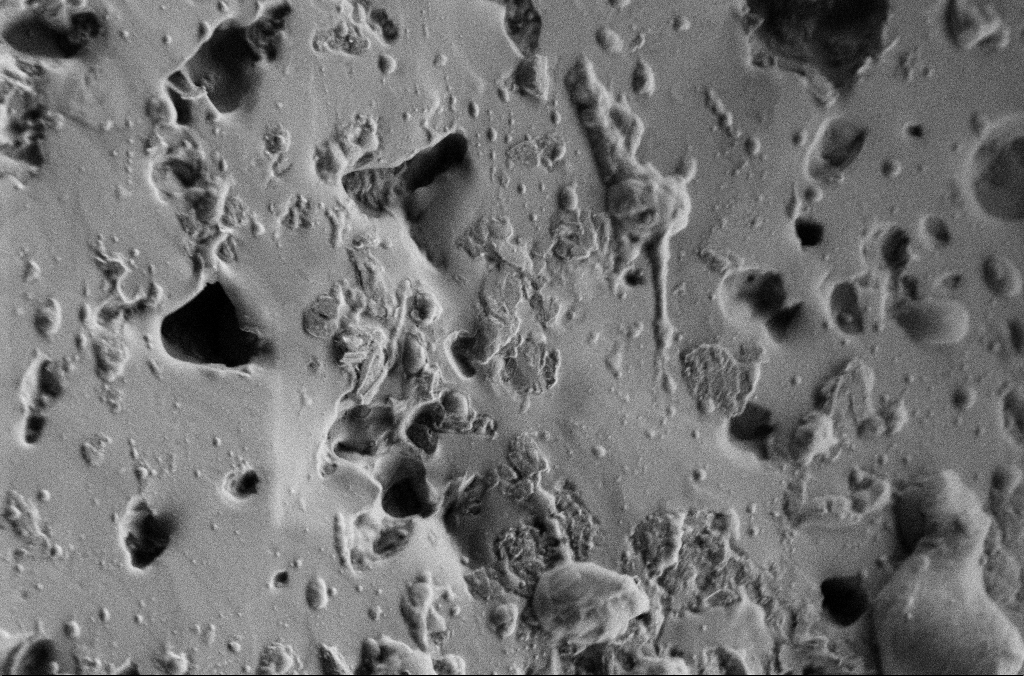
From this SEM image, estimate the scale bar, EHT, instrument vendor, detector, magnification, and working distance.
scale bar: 2000 nm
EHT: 2 kV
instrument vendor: Zeiss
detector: SE2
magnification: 10 K X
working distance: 3 mm